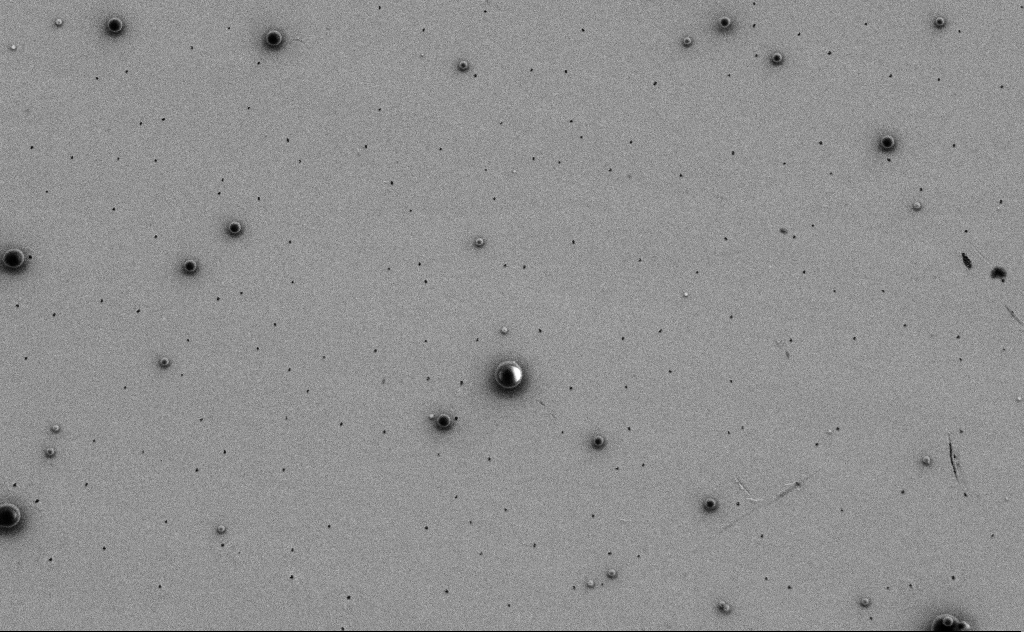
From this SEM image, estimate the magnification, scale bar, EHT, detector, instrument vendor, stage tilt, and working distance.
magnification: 7.63 K X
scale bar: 2000 nm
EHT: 3 kV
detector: SE2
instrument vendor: Zeiss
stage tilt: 0°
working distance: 10 mm